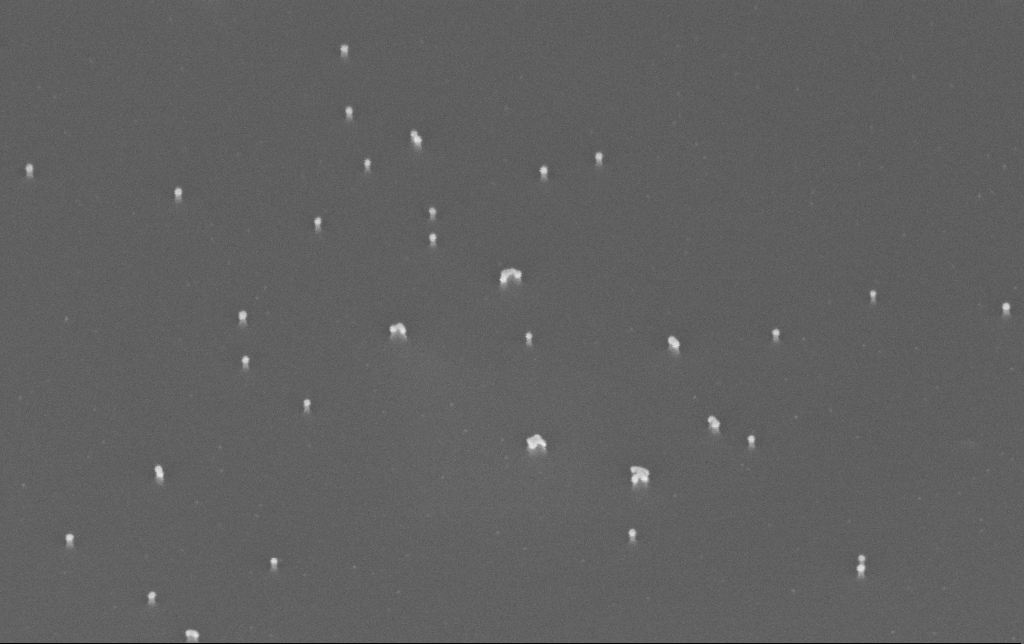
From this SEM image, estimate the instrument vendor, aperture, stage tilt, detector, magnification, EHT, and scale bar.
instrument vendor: Zeiss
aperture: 30 µm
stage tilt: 45°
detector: InLens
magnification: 150 K X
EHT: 10 kV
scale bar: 200 nm